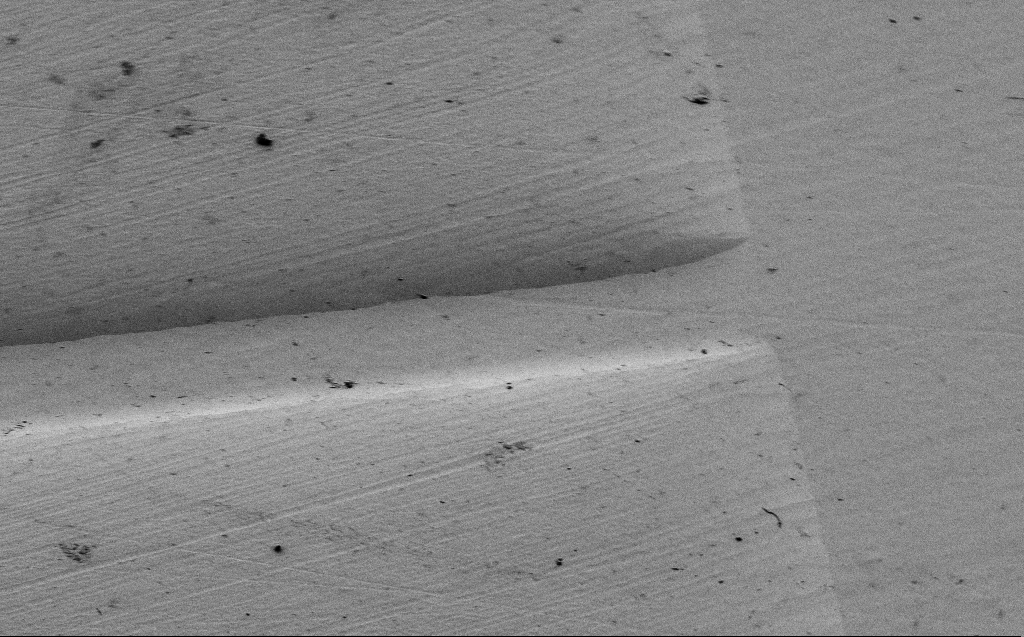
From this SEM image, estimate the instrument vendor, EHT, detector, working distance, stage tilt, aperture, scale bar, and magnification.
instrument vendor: Zeiss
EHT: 1.2 kV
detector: SE2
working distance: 7 mm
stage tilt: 45°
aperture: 30 µm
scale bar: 10000 nm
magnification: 2.97 K X